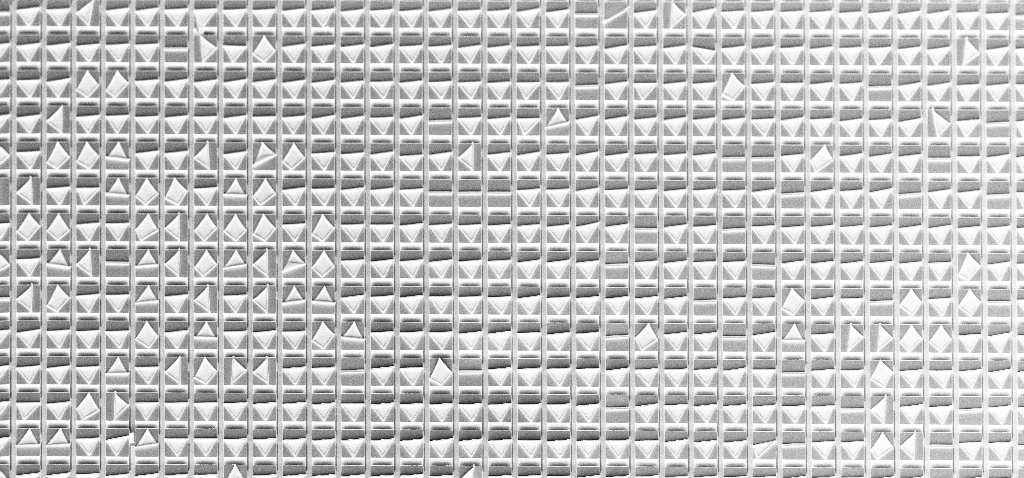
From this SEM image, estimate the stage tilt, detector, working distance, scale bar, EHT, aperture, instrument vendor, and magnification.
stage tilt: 30°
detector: InLens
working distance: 9.5 mm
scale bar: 100000 nm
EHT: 5 kV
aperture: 30 µm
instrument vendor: Zeiss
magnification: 0.541 K X